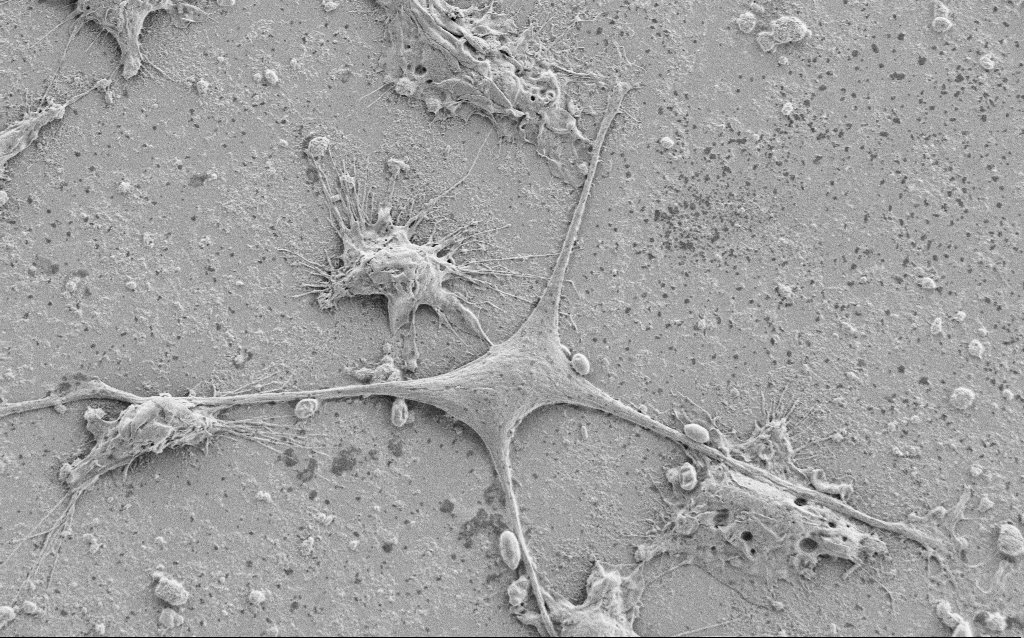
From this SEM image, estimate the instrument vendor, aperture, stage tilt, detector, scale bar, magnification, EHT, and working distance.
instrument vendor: Zeiss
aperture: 30 µm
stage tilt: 0°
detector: SE2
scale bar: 10000 nm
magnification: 2.5 K X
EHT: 1.5 kV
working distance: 6.8 mm